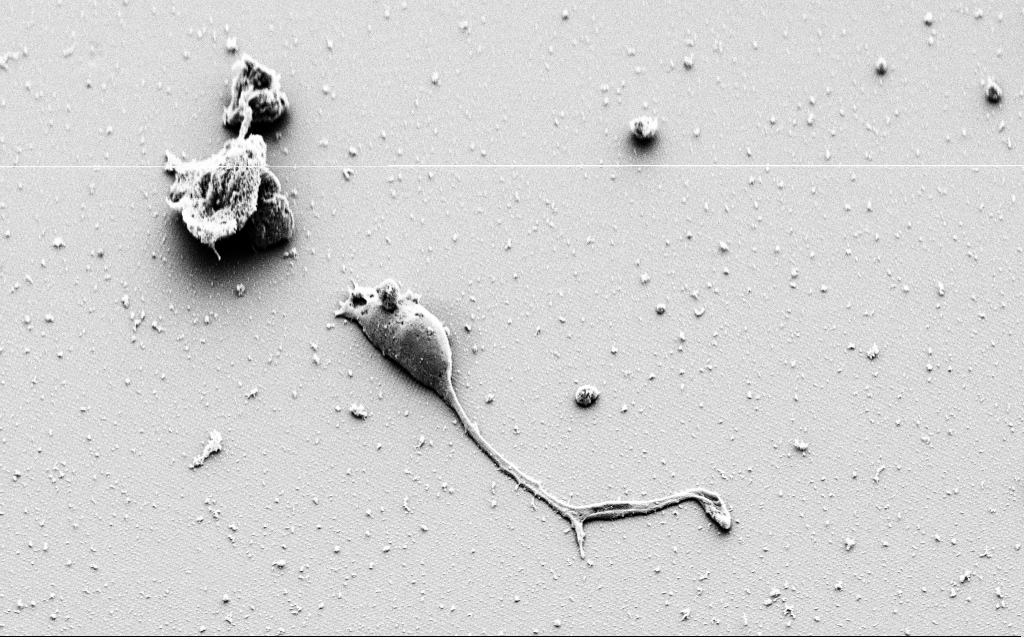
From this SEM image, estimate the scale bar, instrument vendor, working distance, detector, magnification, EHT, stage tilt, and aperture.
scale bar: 20000 nm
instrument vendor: Zeiss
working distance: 9 mm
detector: SE2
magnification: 3.12 K X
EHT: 3 kV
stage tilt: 45°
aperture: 30 µm